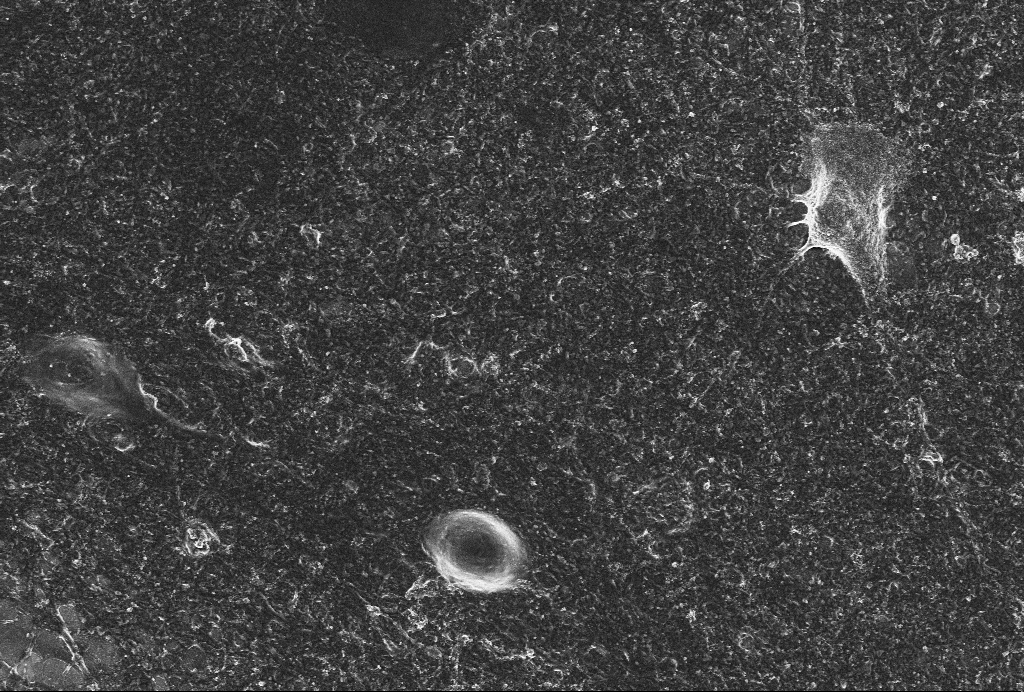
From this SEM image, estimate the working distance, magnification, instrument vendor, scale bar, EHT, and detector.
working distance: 6 mm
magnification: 3 K X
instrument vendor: Zeiss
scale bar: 10000 nm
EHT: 4 kV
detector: SE2